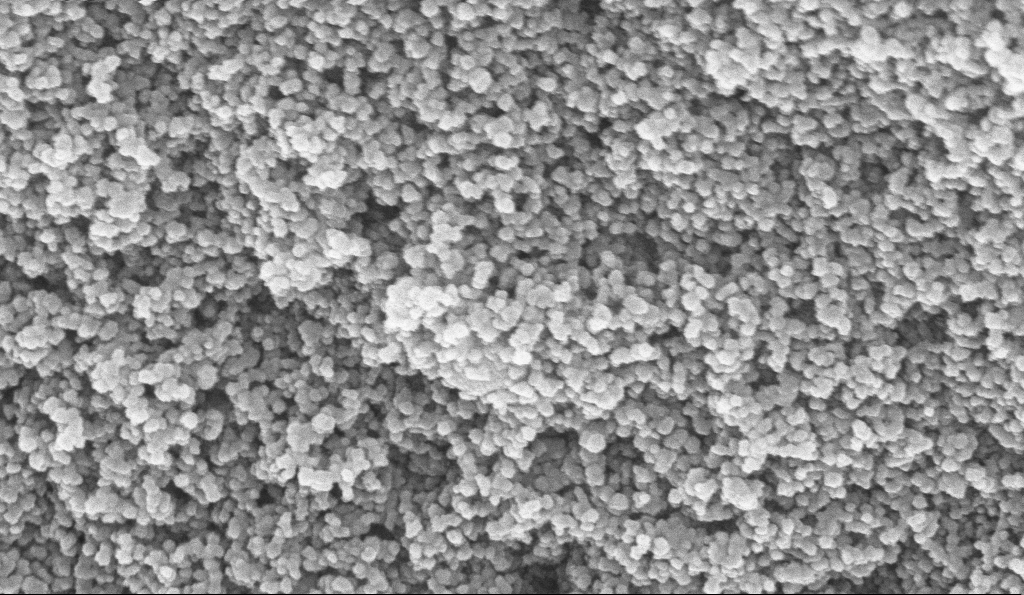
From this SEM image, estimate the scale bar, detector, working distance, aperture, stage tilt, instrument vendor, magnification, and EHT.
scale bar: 200 nm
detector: InLens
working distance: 6 mm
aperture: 30 µm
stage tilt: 0°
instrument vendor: Zeiss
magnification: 135 K X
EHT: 10 kV